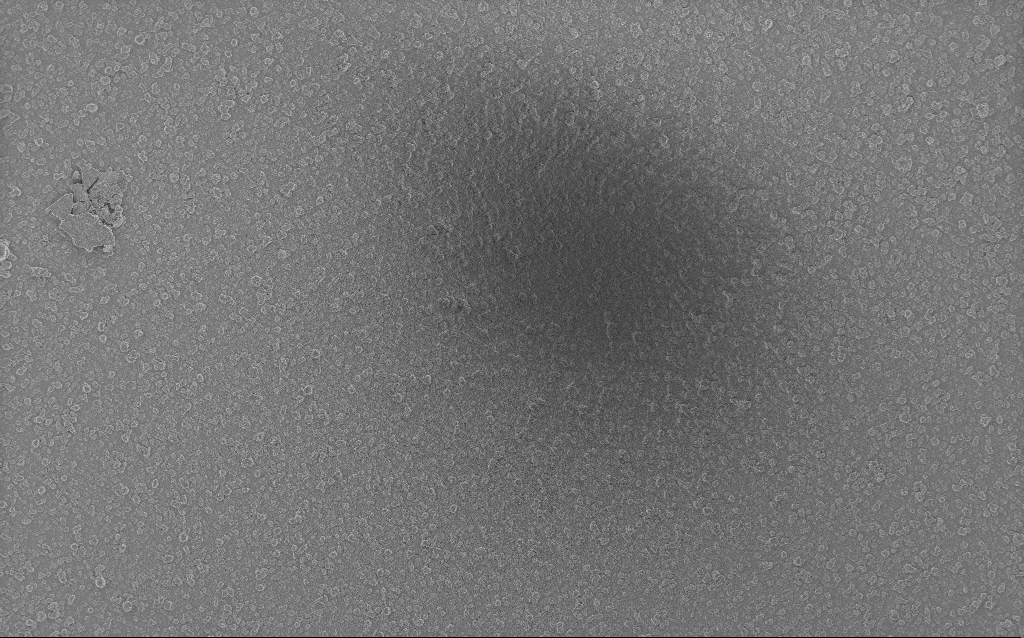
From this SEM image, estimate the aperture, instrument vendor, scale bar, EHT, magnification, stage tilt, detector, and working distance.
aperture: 20 µm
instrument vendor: Zeiss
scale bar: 200000 nm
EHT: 5 kV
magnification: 0.235 K X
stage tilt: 0°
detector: InLens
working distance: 2.6 mm